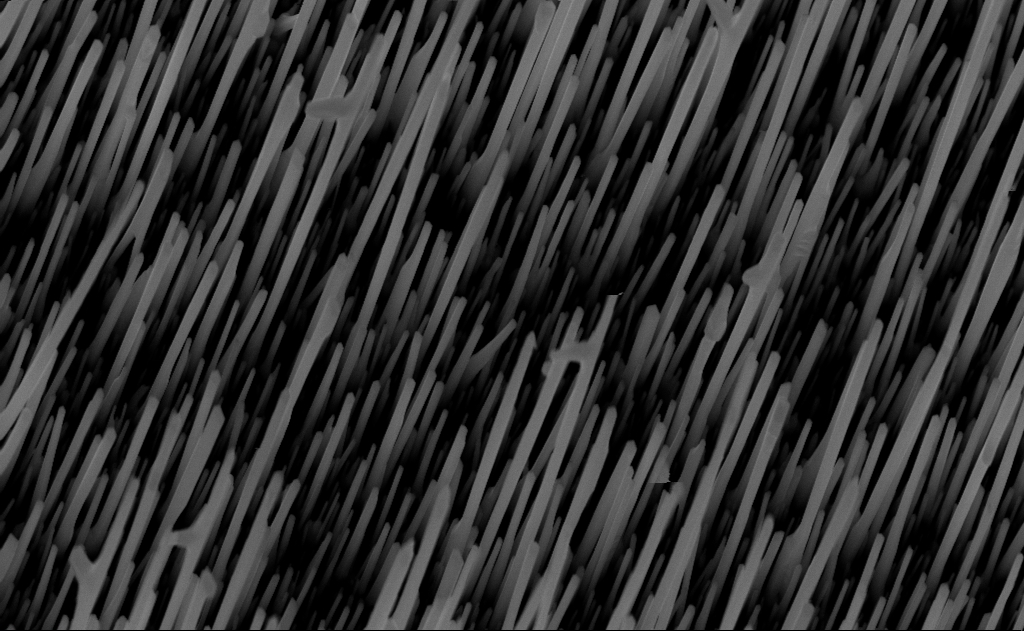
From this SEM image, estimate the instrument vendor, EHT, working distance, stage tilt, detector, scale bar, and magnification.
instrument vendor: Zeiss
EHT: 10 kV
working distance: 8 mm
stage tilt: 0°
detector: InLens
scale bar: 1000 nm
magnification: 40 K X